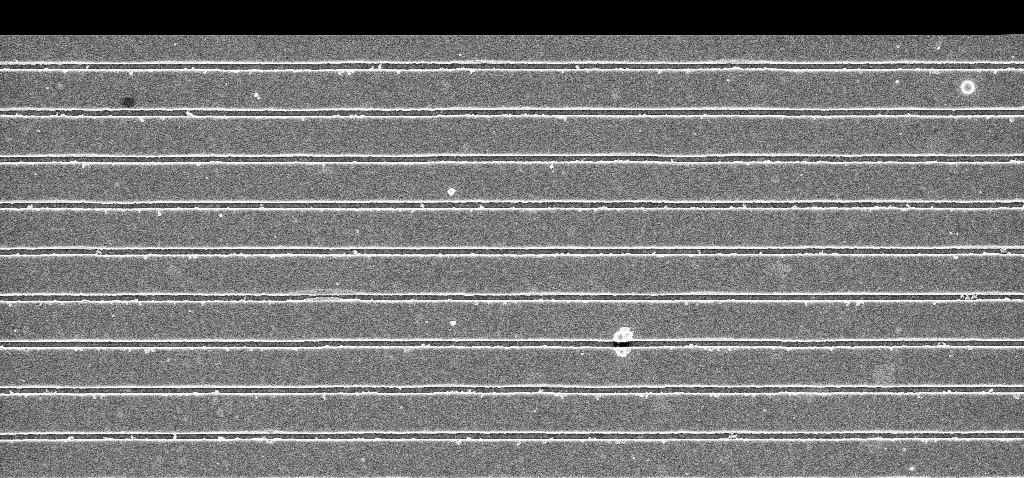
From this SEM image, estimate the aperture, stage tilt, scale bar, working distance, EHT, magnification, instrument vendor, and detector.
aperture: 30 µm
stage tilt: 0°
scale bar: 2000 nm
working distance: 5.3 mm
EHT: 5 kV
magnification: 21.25 K X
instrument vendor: Zeiss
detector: InLens